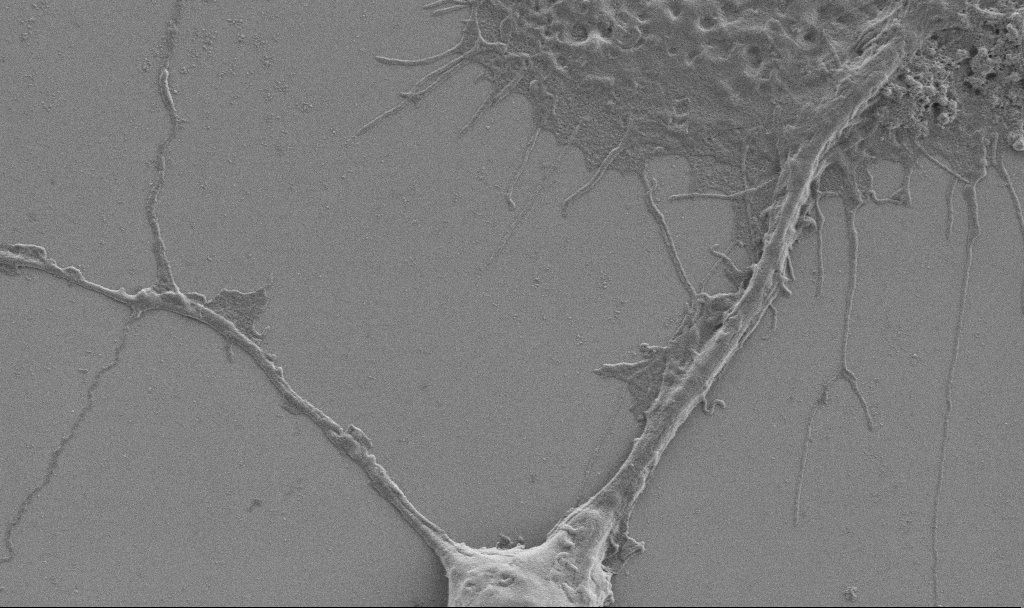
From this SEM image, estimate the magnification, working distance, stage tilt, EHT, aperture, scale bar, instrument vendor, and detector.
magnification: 10 K X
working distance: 6.9 mm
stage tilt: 0°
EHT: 0.9 kV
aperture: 30 µm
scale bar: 2000 nm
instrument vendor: Zeiss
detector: SE2